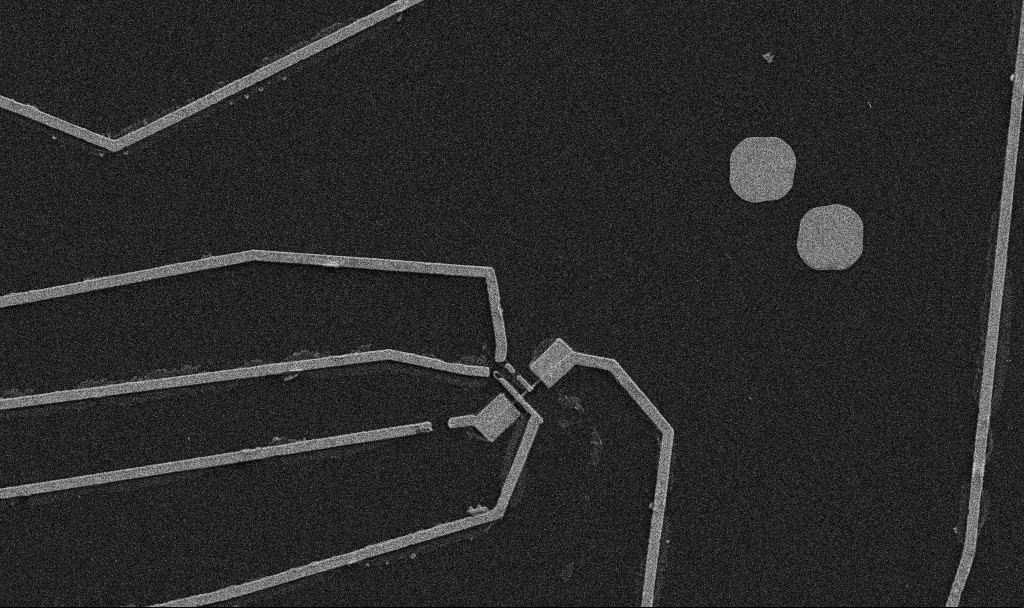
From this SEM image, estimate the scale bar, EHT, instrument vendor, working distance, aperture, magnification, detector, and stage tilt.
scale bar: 10000 nm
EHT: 5 kV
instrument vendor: Zeiss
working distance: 10.7 mm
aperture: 30 µm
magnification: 5 K X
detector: SE2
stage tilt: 0°